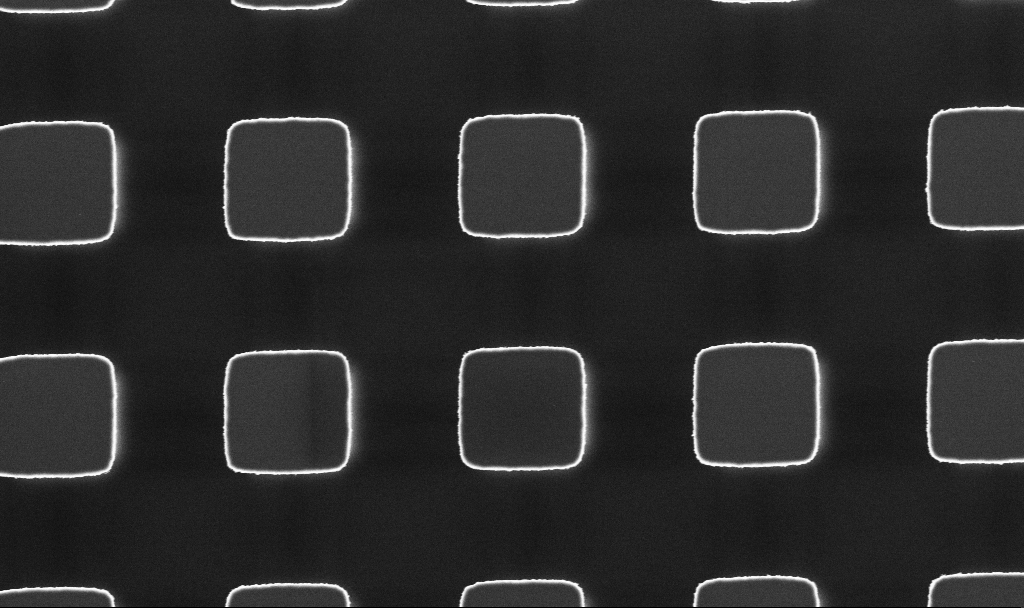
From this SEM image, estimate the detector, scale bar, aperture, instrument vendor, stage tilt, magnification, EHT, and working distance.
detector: InLens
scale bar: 2000 nm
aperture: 30 µm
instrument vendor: Zeiss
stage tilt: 0°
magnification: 10.71 K X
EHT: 3 kV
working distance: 7.8 mm